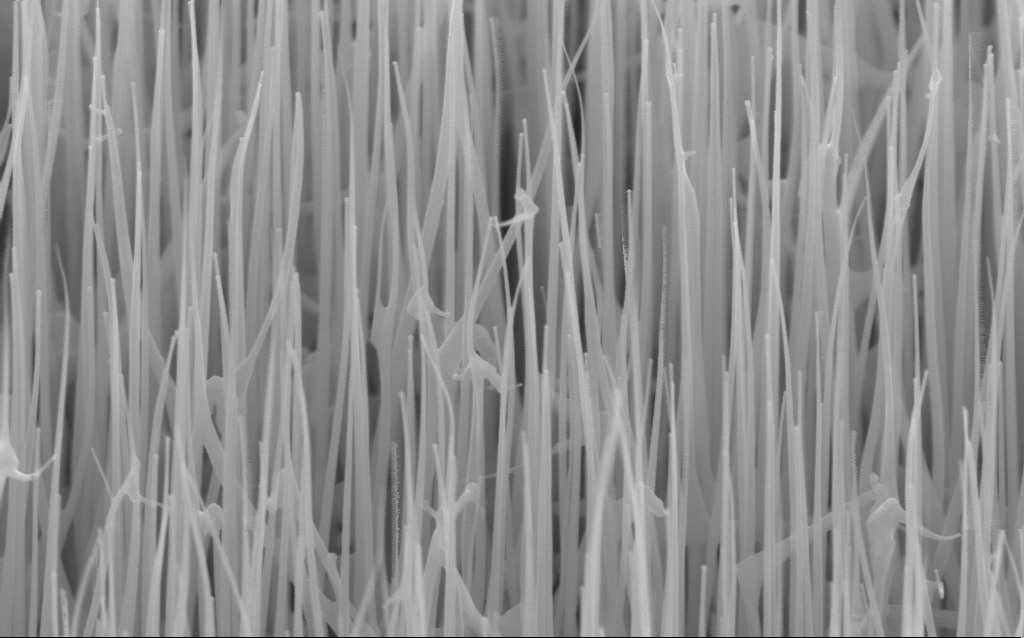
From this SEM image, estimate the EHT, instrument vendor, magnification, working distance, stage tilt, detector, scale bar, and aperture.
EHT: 10 kV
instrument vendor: Zeiss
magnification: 80 K X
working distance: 6 mm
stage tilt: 45°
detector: InLens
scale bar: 200 nm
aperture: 30 µm